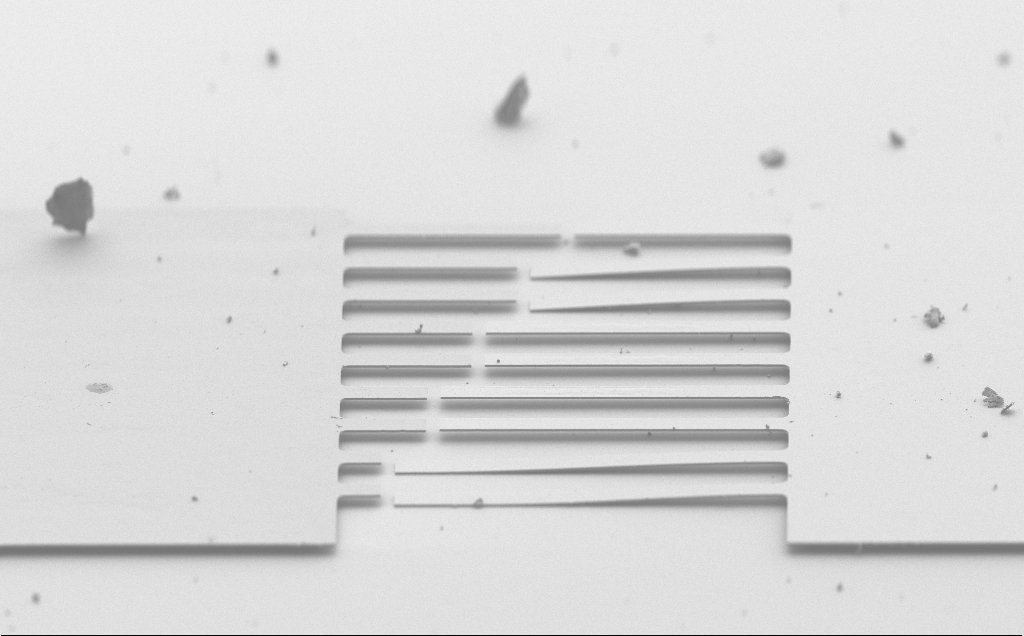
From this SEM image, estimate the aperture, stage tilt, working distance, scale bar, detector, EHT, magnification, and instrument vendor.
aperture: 30 µm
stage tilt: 70°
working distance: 5 mm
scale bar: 100000 nm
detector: SE2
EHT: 3 kV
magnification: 0.651 K X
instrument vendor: Zeiss